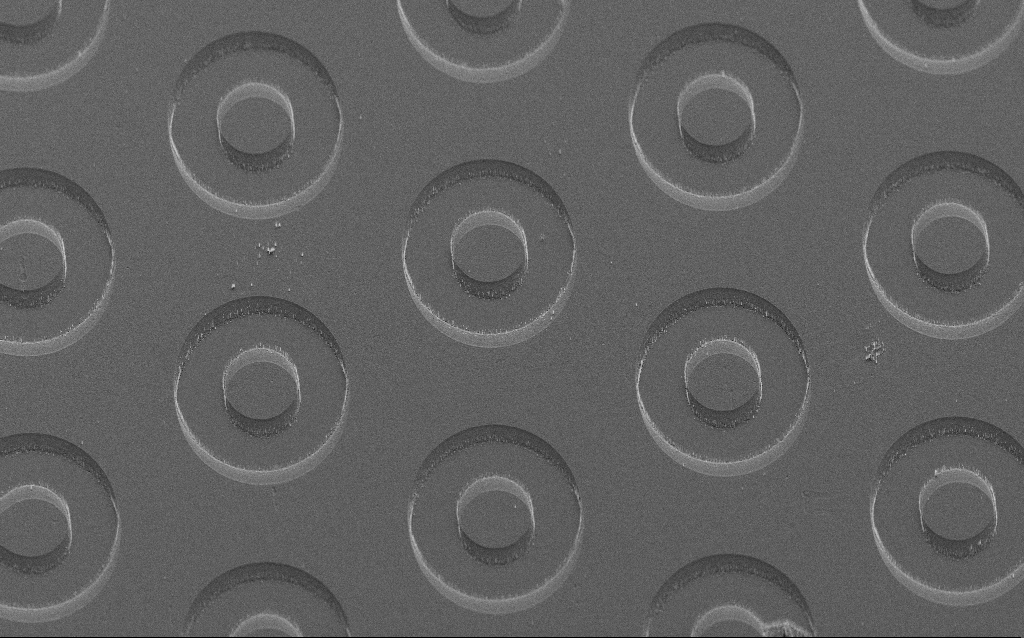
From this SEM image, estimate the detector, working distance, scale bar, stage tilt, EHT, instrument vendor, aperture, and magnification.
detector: SE2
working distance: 8 mm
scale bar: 100000 nm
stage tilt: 45°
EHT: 5 kV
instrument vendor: Zeiss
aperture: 30 µm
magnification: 0.704 K X